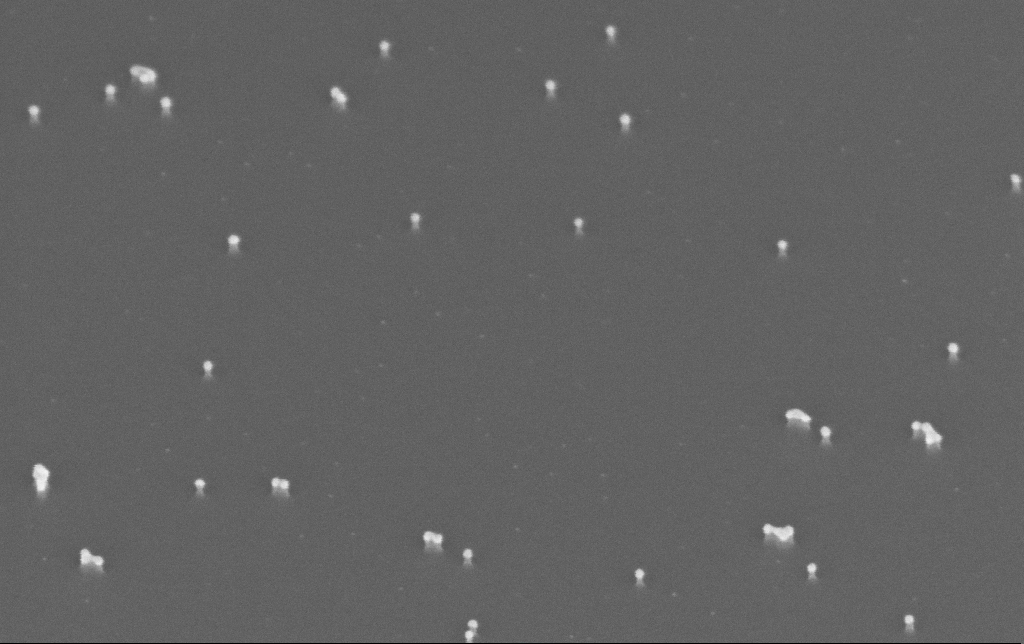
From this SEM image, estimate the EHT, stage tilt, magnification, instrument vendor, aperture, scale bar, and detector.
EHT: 10 kV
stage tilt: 45°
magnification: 200 K X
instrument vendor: Zeiss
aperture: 30 µm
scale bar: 100 nm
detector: InLens